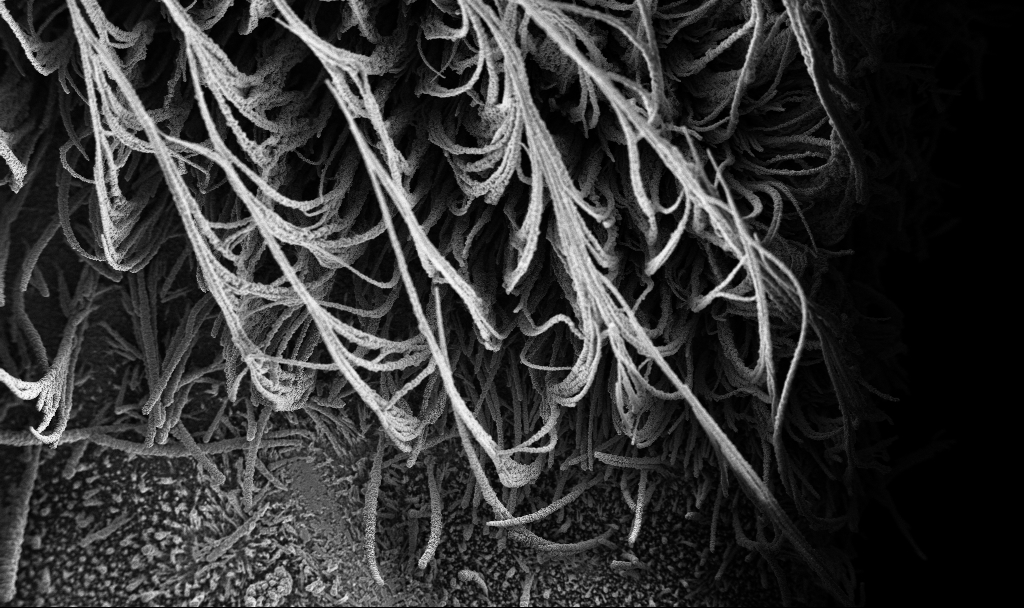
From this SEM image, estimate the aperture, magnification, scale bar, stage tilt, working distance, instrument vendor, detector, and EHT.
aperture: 30 µm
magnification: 0.15 K X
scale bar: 100000 nm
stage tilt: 0°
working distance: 3.7 mm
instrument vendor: Zeiss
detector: InLens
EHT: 3 kV